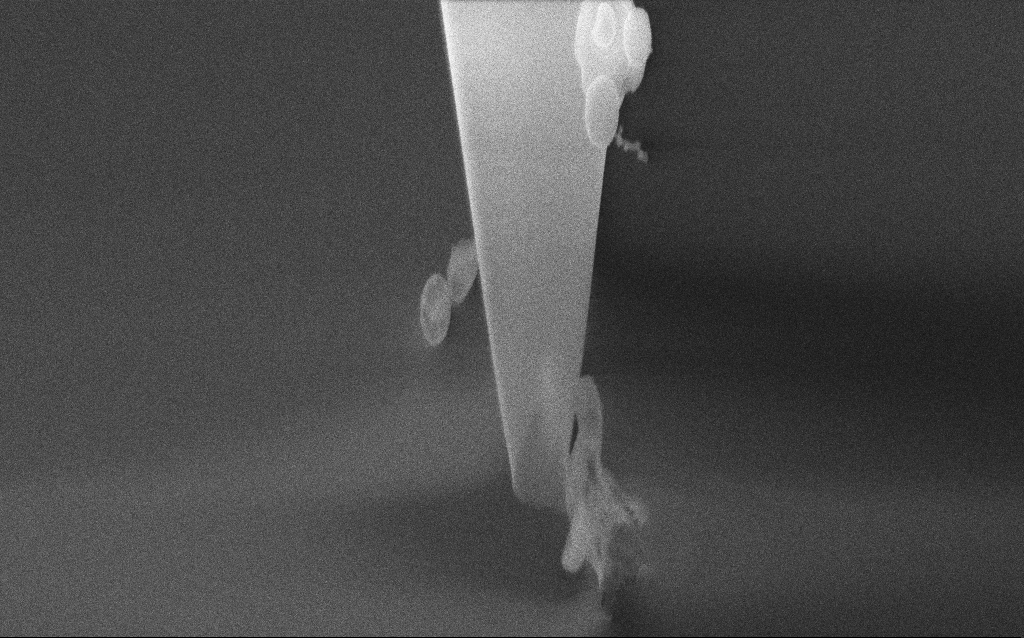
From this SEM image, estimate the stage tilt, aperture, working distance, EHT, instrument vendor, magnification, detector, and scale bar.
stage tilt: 45°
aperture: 30 µm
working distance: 4 mm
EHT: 5 kV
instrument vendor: Zeiss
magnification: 56.06 K X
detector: InLens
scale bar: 1000 nm